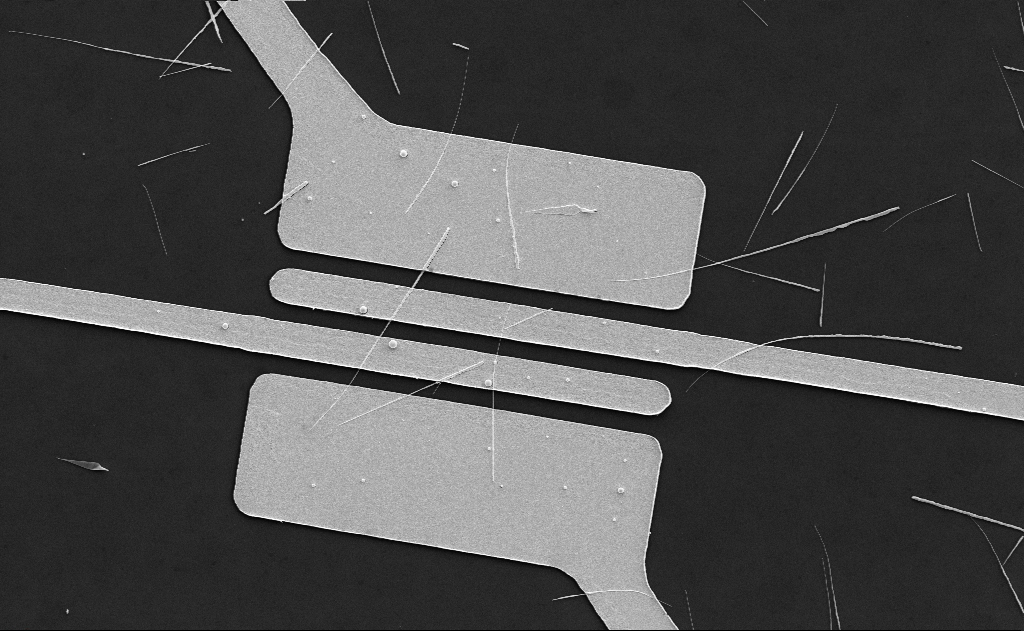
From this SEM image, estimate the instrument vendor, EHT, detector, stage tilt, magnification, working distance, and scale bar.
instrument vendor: Zeiss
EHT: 5 kV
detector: SE2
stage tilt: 0°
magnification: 5 K X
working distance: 19 mm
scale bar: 10000 nm